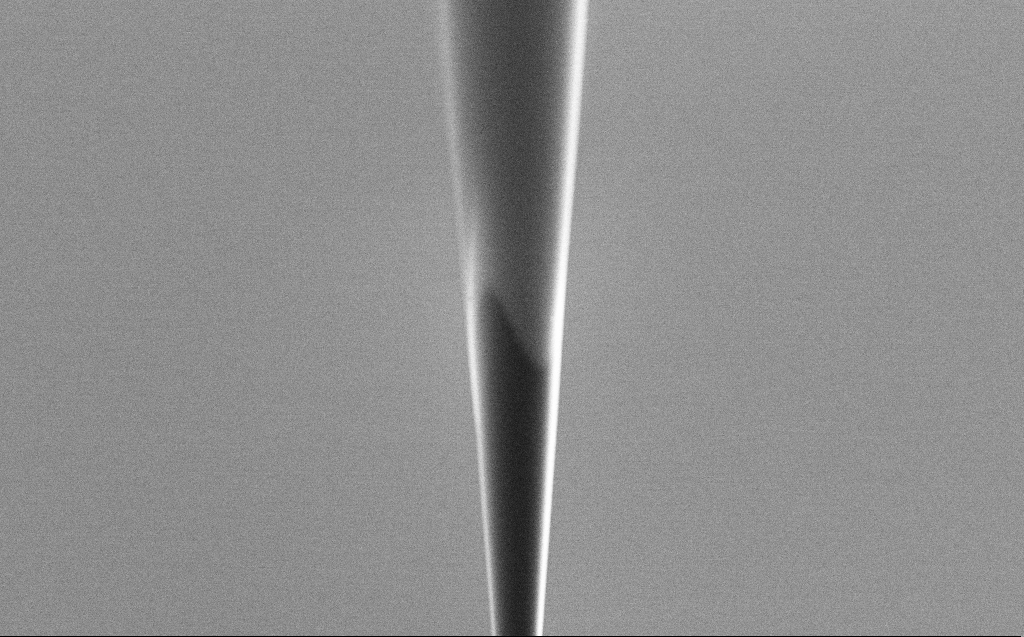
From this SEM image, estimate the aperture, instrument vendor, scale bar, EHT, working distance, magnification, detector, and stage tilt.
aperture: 30 µm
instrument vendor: Zeiss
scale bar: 10000 nm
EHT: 2 kV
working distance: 6 mm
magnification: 5 K X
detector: SE2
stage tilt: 45°